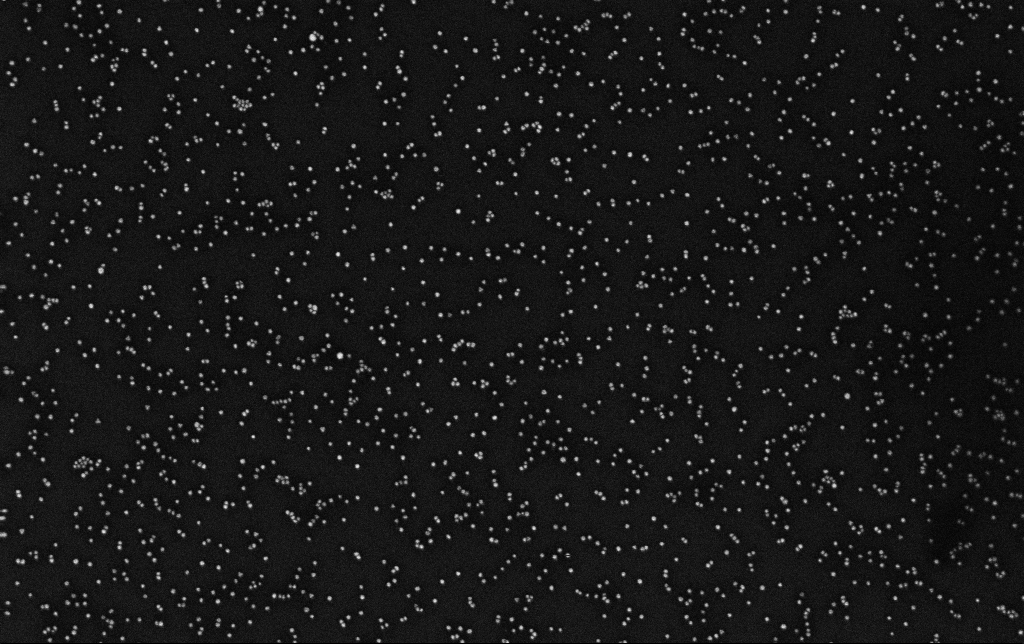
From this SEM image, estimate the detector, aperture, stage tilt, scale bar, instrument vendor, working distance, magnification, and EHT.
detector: InLens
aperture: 30 µm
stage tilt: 0°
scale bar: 200 nm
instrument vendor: Zeiss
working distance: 3.3 mm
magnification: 100 K X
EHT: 10 kV